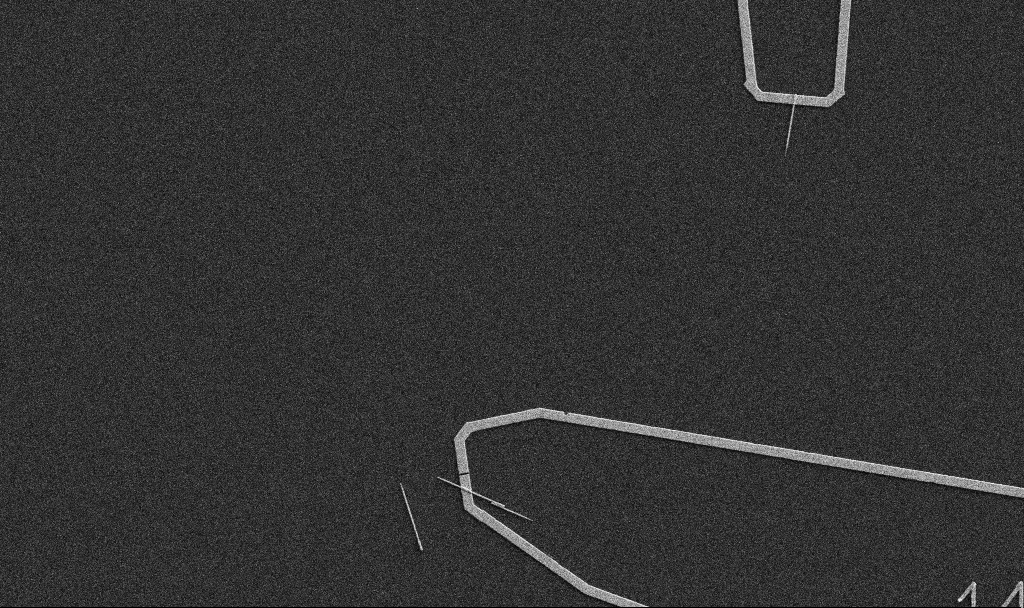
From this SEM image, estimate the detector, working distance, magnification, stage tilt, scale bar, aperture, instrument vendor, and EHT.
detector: SE2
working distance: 10.7 mm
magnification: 5 K X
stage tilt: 0°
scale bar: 10000 nm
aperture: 30 µm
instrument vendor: Zeiss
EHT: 5 kV